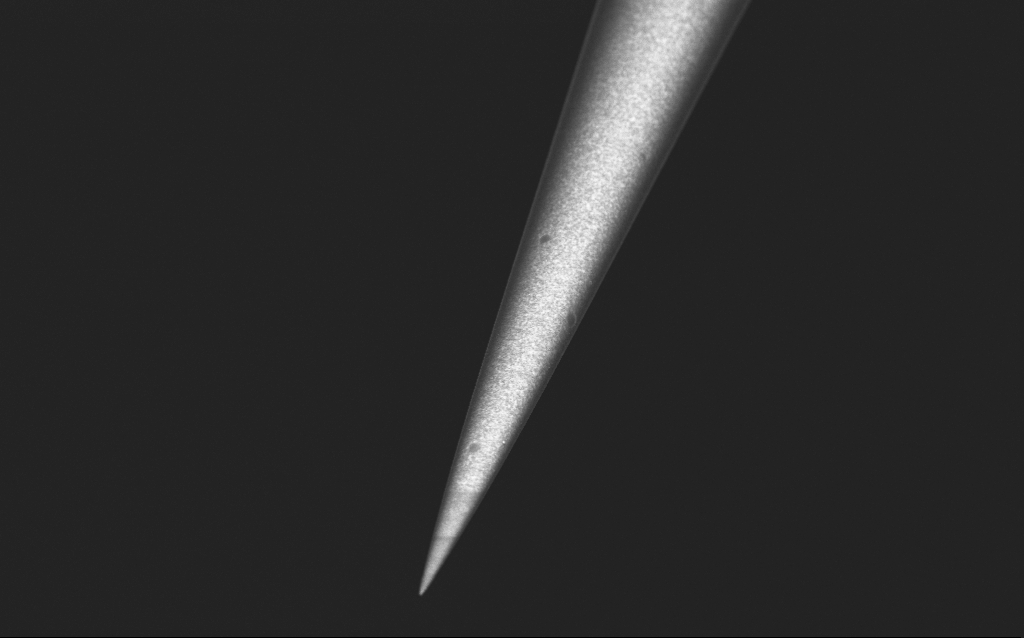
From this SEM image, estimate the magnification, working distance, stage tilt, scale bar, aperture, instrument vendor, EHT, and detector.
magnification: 10 K X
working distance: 5 mm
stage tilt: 45°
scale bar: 2000 nm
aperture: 30 µm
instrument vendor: Zeiss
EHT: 2.5 kV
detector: InLens